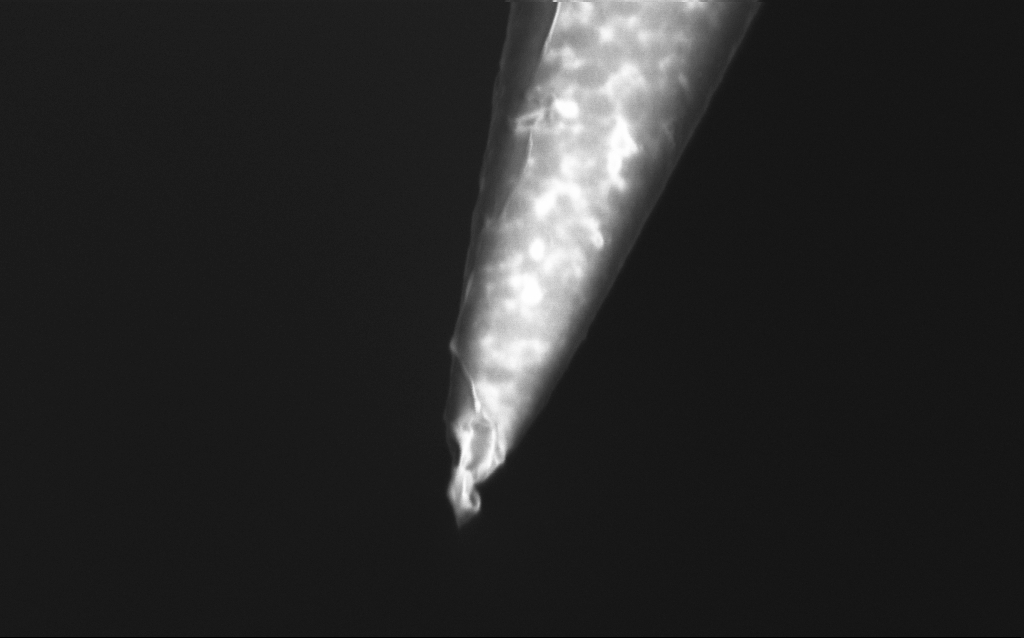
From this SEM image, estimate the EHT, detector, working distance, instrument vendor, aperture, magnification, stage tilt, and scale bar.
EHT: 1 kV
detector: InLens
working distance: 6 mm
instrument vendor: Zeiss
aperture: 30 µm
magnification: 75 K X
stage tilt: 45°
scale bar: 200 nm